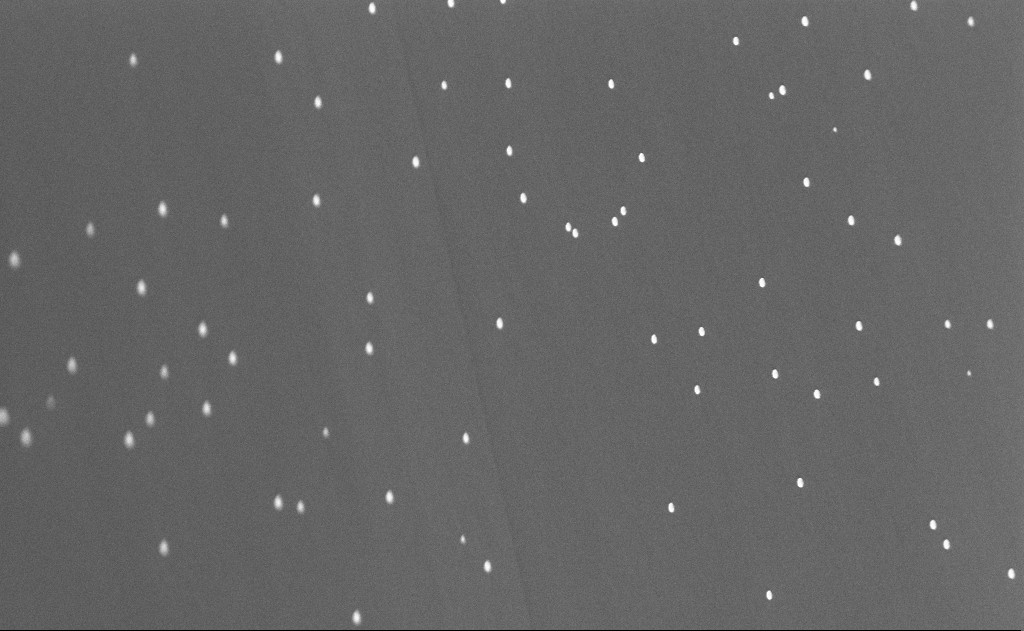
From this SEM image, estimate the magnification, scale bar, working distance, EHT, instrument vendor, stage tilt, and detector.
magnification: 40 K X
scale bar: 1000 nm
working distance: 10 mm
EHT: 10 kV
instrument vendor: Zeiss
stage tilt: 0°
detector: InLens